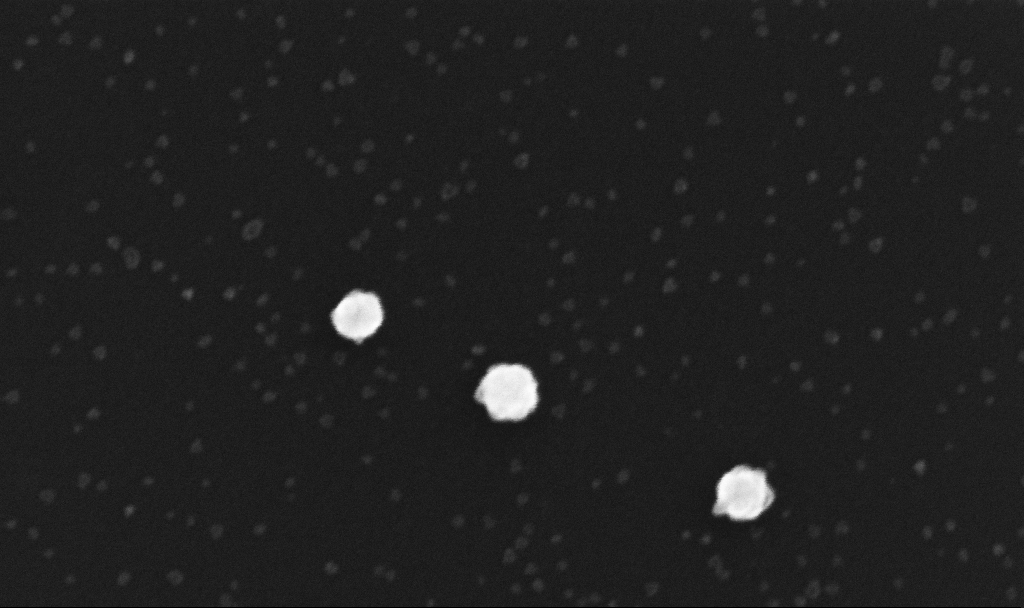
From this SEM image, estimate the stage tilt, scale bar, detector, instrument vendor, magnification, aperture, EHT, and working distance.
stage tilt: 0°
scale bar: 100 nm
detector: InLens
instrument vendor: Zeiss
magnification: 300 K X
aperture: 30 µm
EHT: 10 kV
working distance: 3.4 mm